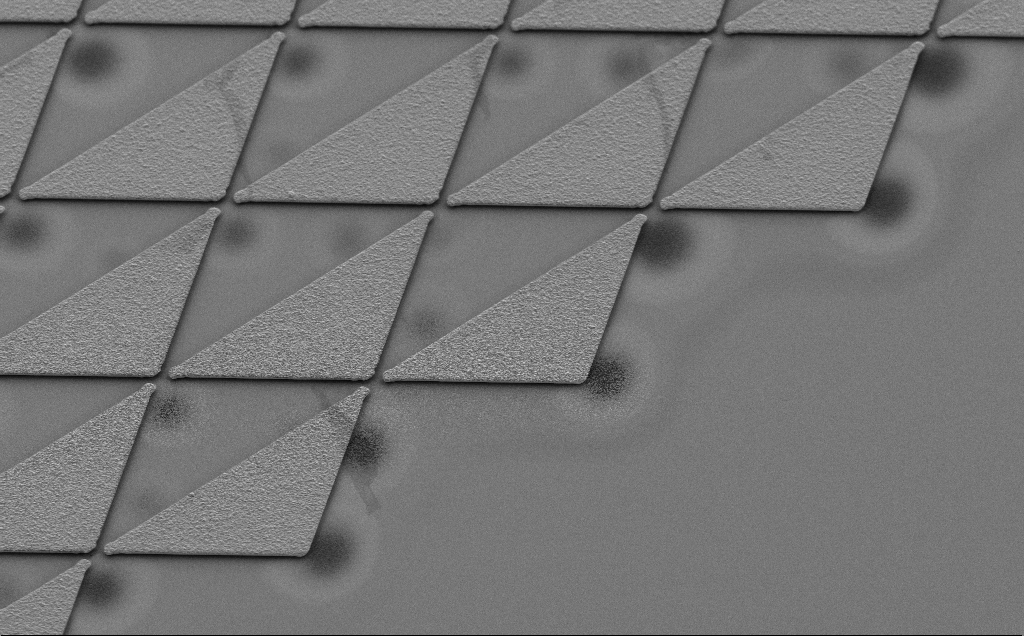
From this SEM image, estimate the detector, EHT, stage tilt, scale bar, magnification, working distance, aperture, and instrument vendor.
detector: SE2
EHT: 5 kV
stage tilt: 30°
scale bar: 10000 nm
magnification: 1.33 K X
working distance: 9 mm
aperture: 30 µm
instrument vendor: Zeiss